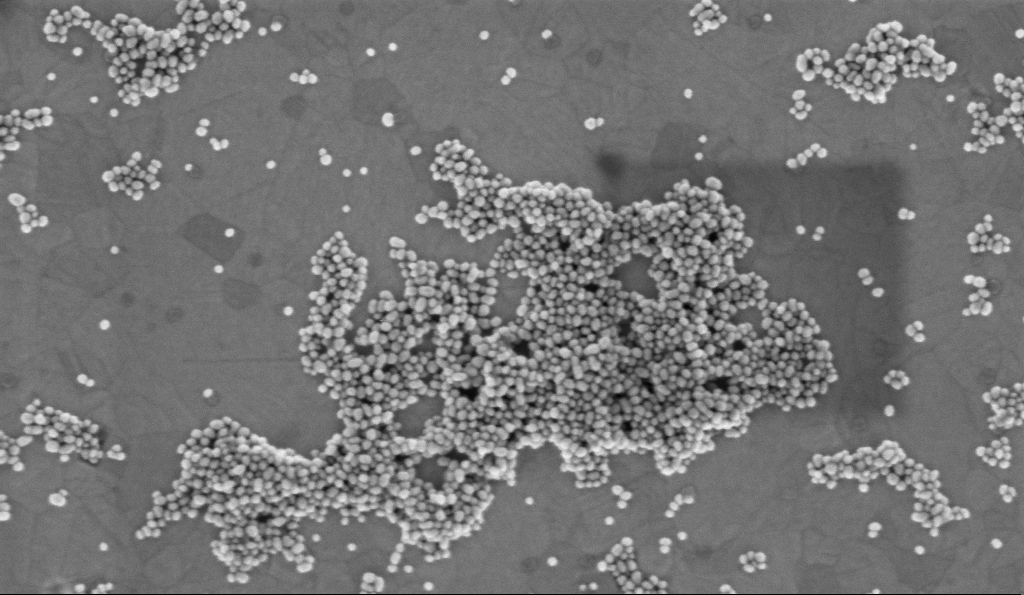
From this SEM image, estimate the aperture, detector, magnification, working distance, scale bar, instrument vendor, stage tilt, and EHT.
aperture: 30 µm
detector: InLens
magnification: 150 K X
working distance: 3.2 mm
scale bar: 100 nm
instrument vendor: Zeiss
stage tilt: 0°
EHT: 2 kV